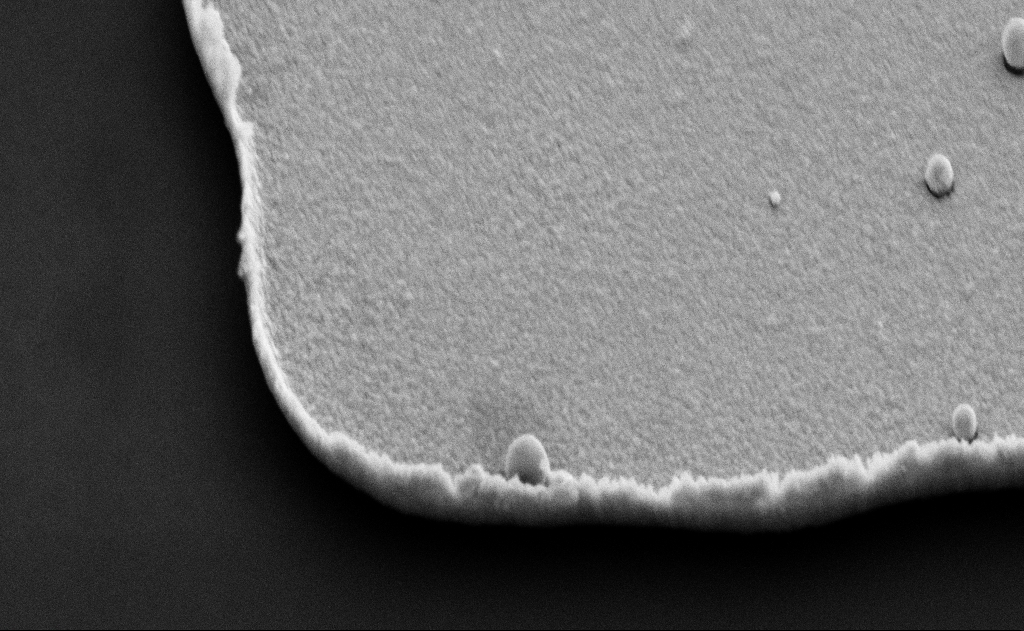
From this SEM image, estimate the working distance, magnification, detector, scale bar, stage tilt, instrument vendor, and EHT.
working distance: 10 mm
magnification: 48.14 K X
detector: SE2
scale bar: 1000 nm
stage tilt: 45°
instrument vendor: Zeiss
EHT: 5 kV